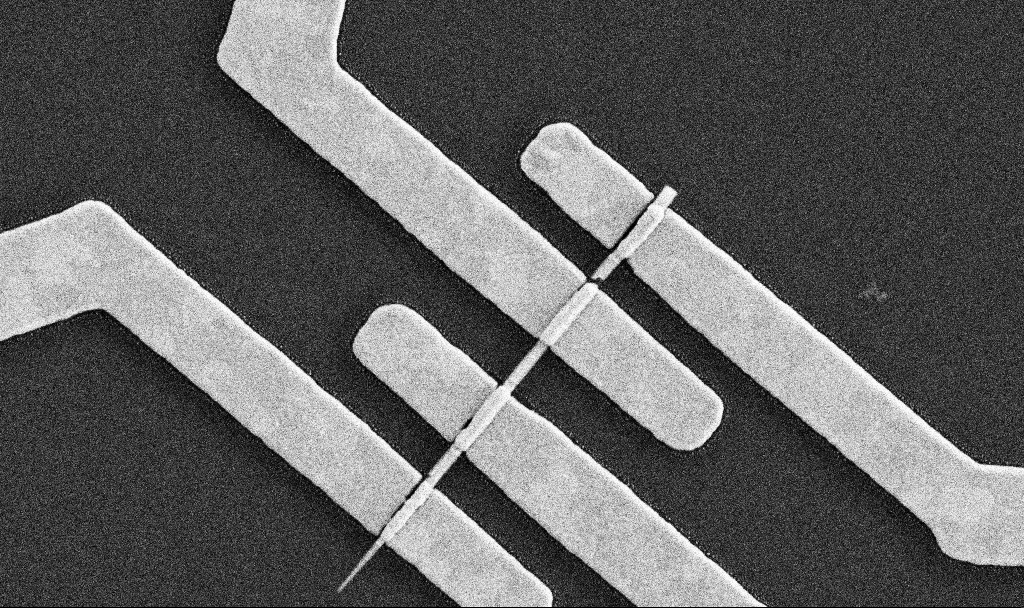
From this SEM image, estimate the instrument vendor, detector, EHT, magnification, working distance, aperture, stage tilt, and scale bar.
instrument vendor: Zeiss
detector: SE2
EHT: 5 kV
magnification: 43.62 K X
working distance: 7.6 mm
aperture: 30 µm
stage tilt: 0°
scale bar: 1000 nm